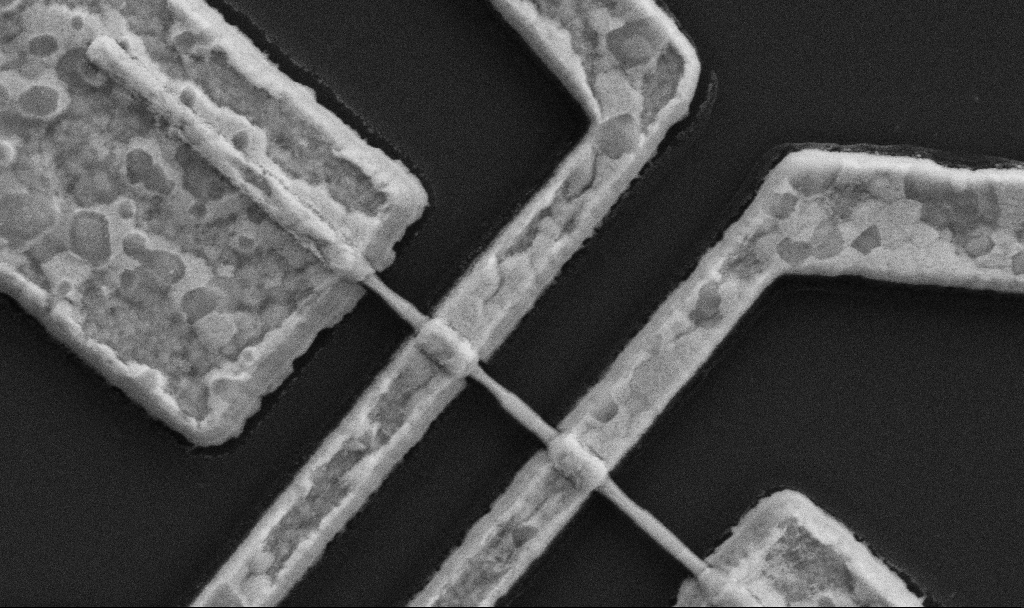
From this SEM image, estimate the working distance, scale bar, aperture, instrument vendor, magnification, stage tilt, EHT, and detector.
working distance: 10.7 mm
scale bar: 1000 nm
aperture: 30 µm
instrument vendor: Zeiss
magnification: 60 K X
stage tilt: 0°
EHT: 5 kV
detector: SE2